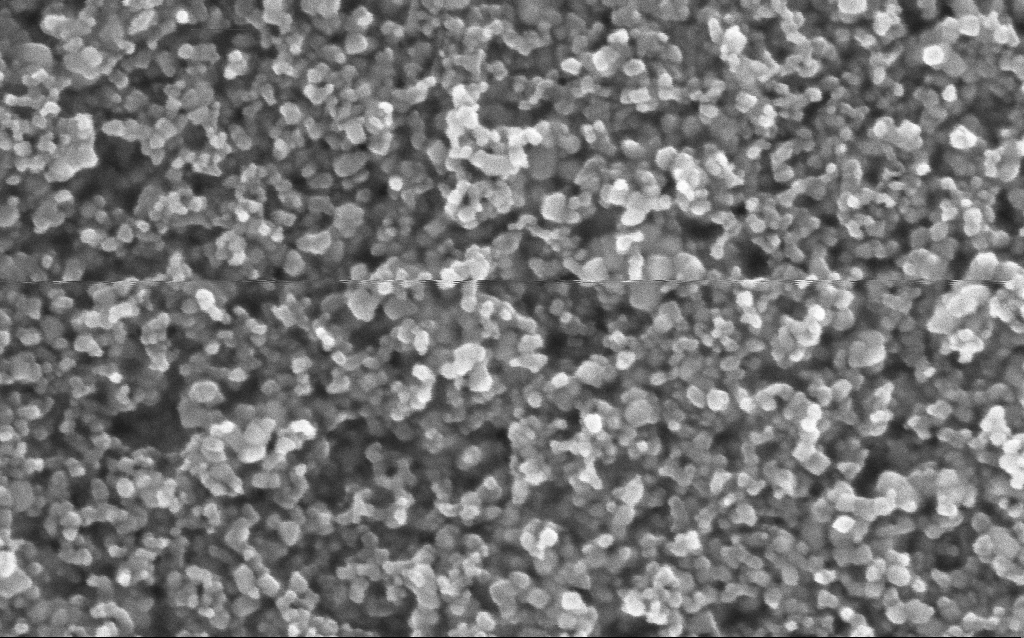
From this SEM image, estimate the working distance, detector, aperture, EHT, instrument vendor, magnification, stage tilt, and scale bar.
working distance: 6.4 mm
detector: InLens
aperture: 30 µm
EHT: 5 kV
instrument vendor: Zeiss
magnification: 211.33 K X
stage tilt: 0°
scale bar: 100 nm